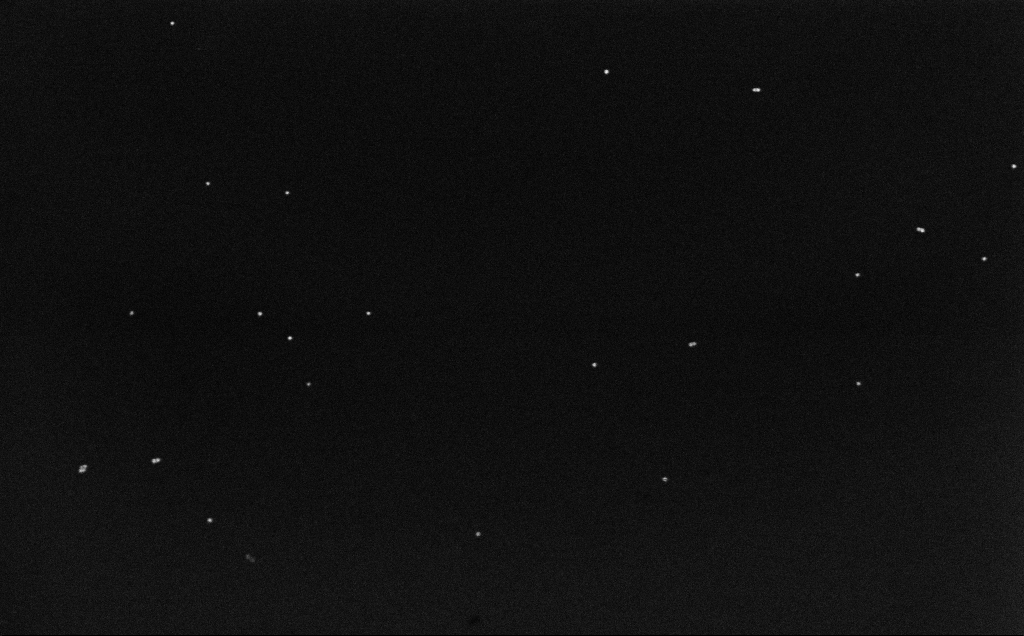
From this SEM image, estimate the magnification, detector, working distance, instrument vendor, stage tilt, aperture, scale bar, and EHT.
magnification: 100 K X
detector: InLens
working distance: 6.6 mm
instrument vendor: Zeiss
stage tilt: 0°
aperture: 30 µm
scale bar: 200 nm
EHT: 10 kV